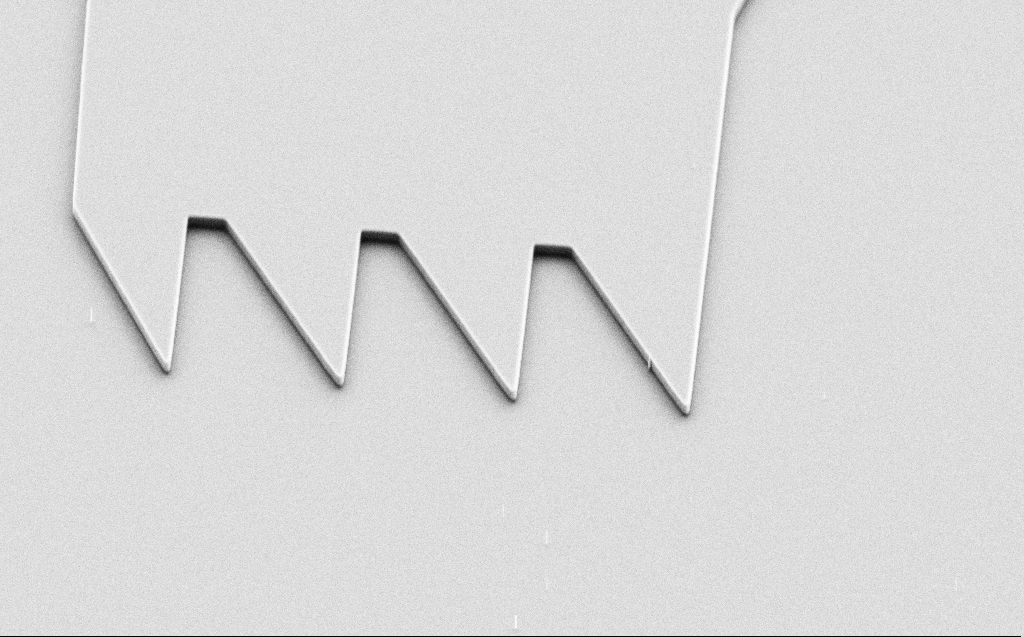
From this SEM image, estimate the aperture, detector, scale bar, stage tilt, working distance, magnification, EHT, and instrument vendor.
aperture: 30 µm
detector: SE2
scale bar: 10000 nm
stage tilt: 45°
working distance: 5 mm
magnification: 5.66 K X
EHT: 5 kV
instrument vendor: Zeiss